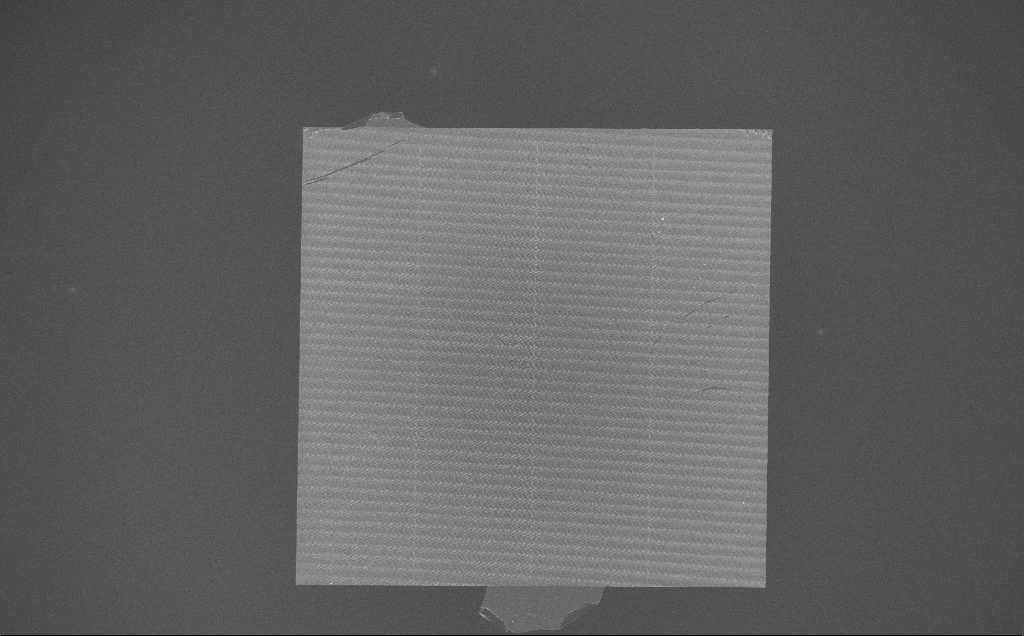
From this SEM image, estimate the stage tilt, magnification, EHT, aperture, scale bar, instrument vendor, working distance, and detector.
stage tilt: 0°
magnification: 0.172 K X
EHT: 10 kV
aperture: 30 µm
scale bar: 100000 nm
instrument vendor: Zeiss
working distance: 7 mm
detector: InLens